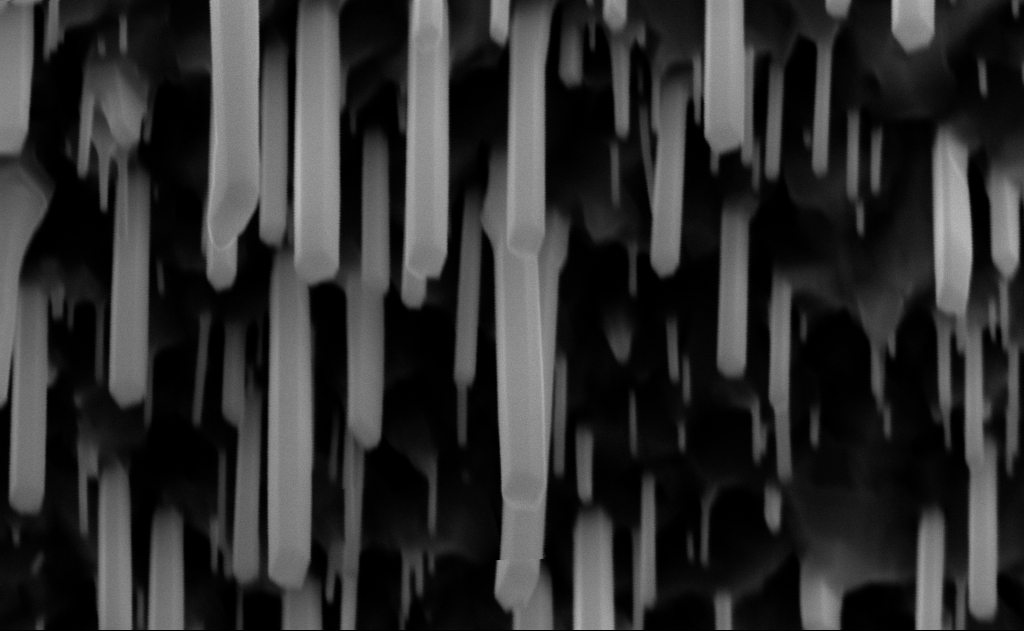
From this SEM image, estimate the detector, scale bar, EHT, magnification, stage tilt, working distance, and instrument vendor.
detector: InLens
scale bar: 200 nm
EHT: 10 kV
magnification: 100 K X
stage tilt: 0°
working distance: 9 mm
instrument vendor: Zeiss